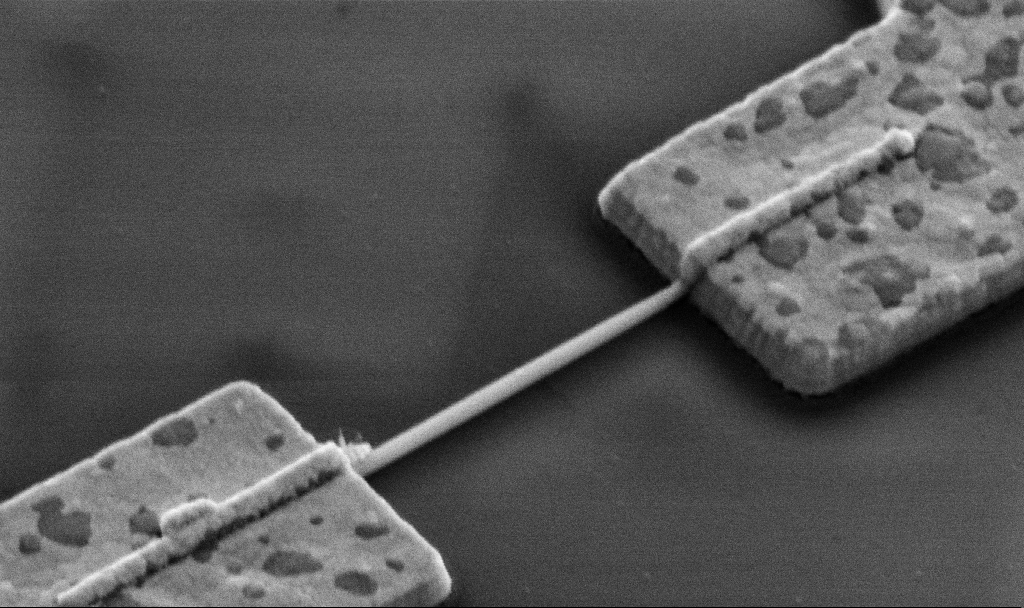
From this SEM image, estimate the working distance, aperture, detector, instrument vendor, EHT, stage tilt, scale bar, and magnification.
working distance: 13.6 mm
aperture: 30 µm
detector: SE2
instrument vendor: Zeiss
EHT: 5 kV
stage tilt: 45°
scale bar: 1000 nm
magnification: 60 K X